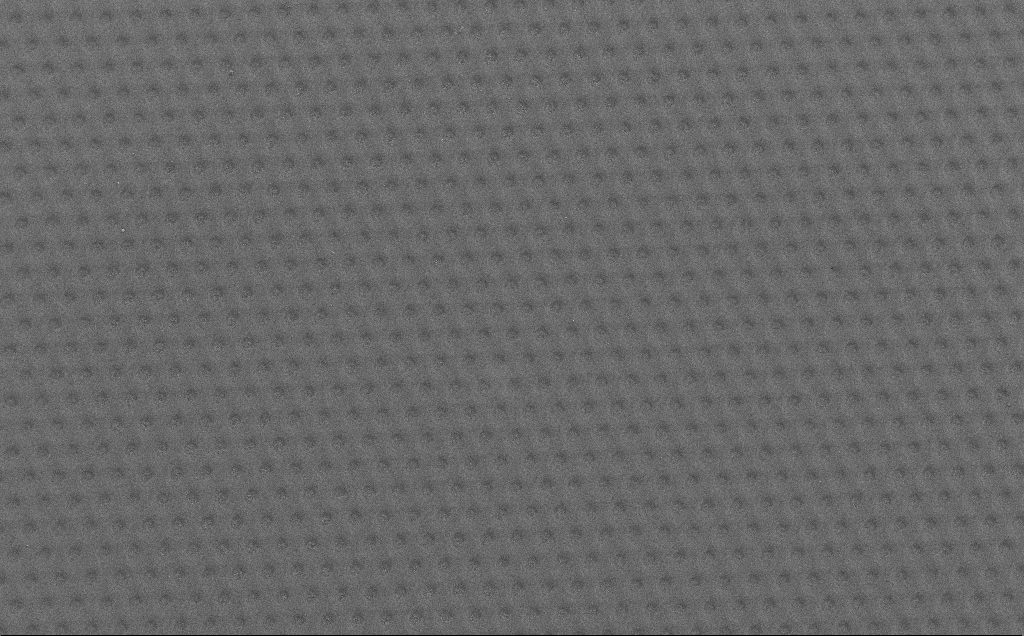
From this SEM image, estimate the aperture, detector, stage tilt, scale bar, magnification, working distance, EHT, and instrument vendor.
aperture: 30 µm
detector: SE2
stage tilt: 0°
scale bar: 100000 nm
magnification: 0.544 K X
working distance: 4 mm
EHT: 1.5 kV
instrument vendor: Zeiss